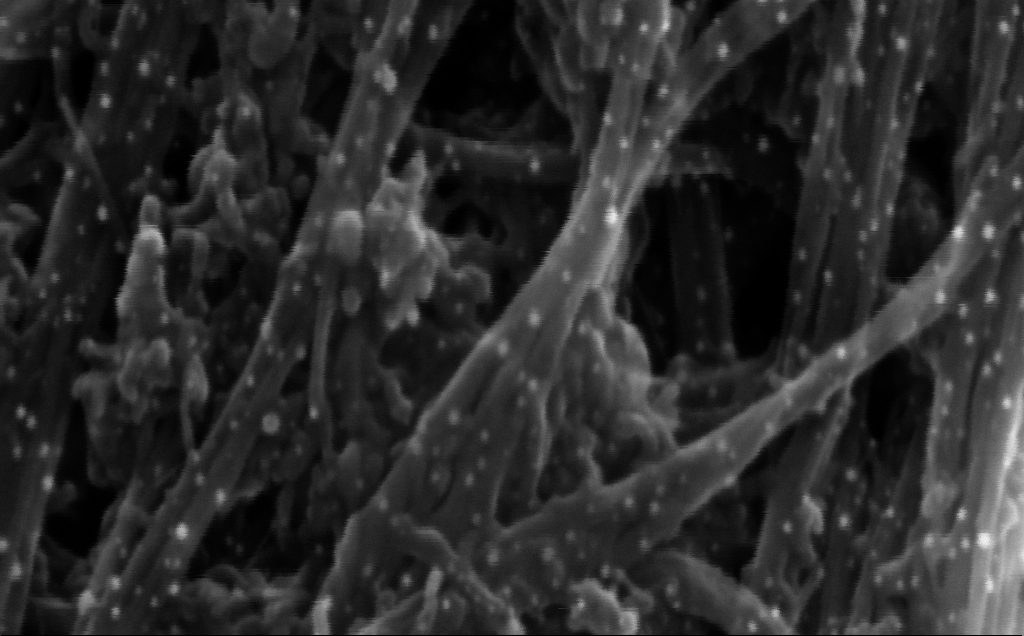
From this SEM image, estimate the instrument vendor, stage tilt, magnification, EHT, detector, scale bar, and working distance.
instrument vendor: Zeiss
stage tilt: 0°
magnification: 588.56 K X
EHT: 5 kV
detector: InLens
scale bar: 100 nm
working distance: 4 mm